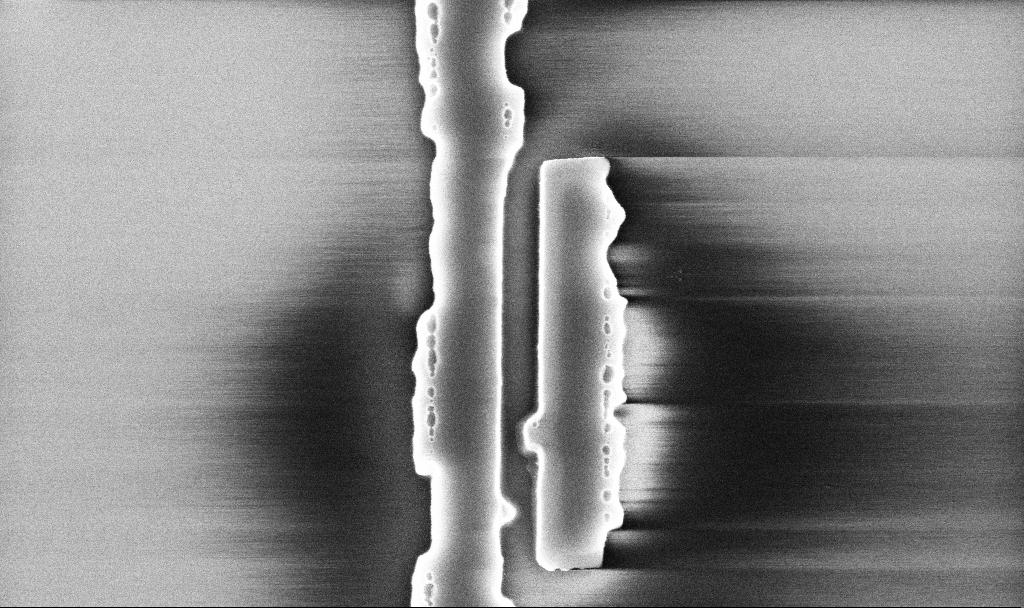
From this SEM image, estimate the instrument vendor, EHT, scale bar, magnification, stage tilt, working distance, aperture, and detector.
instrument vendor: Zeiss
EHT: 5 kV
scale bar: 1000 nm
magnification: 50.01 K X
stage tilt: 0°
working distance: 10.1 mm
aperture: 30 µm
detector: InLens